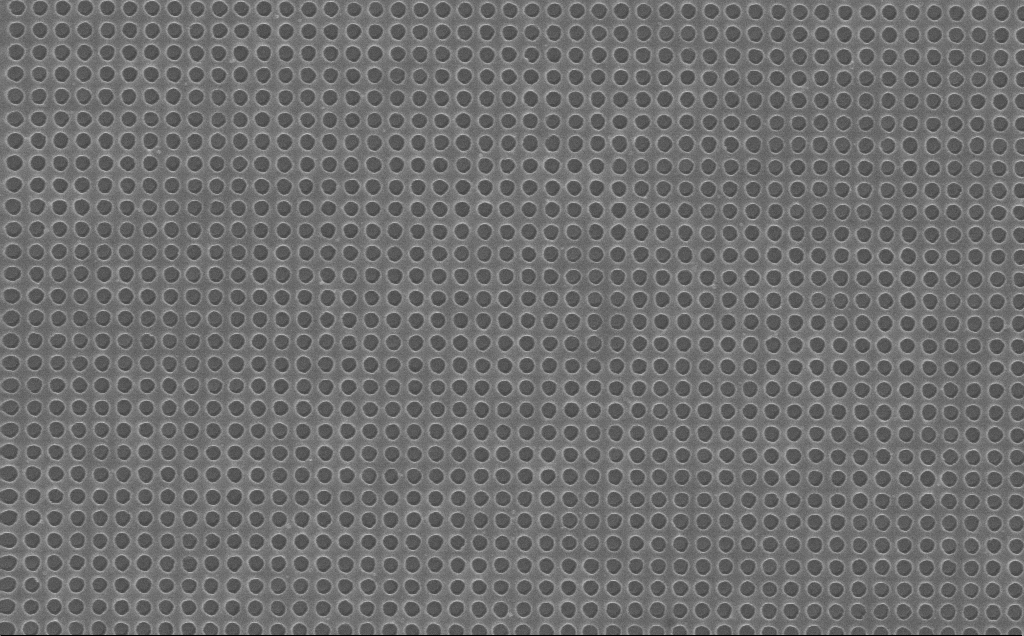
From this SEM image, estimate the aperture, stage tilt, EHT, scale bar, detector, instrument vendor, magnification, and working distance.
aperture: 30 µm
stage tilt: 0°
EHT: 10 kV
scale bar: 1000 nm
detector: InLens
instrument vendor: Zeiss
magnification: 41.1 K X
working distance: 7 mm